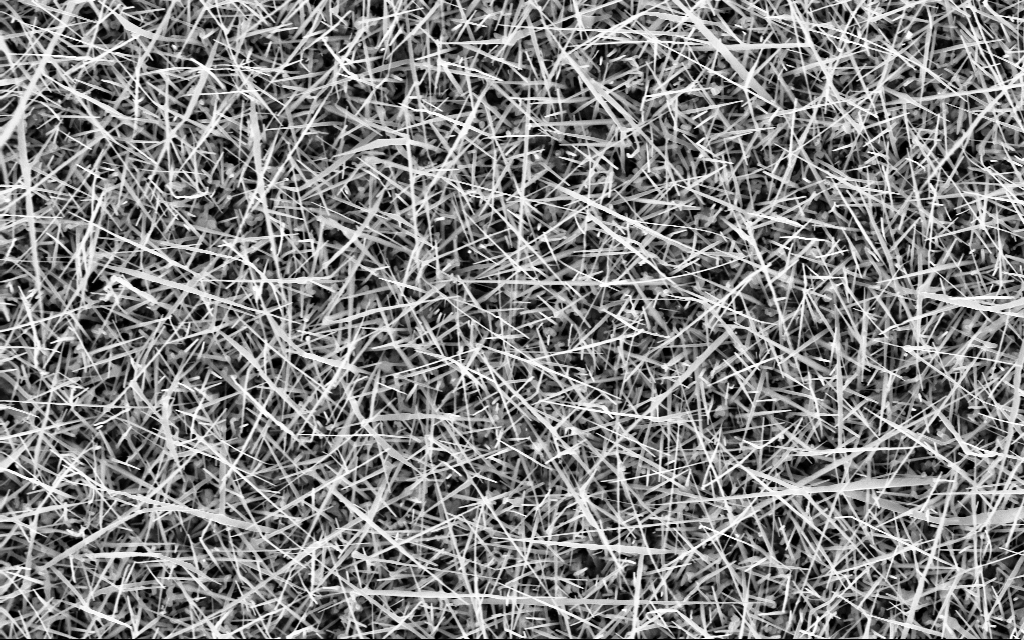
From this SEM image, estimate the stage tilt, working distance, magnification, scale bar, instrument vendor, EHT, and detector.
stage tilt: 0°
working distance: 5 mm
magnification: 20 K X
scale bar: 2000 nm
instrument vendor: Zeiss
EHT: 10 kV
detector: InLens